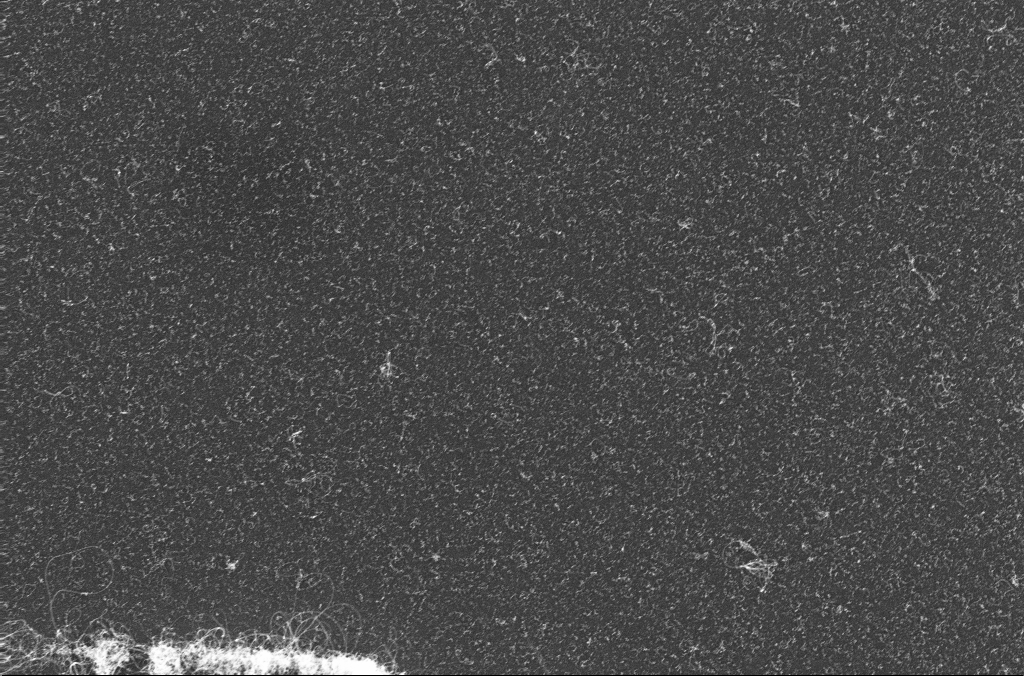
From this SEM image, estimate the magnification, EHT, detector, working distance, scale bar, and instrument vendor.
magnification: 15 K X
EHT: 10 kV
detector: InLens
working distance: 3.3 mm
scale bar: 1000 nm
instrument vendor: Zeiss